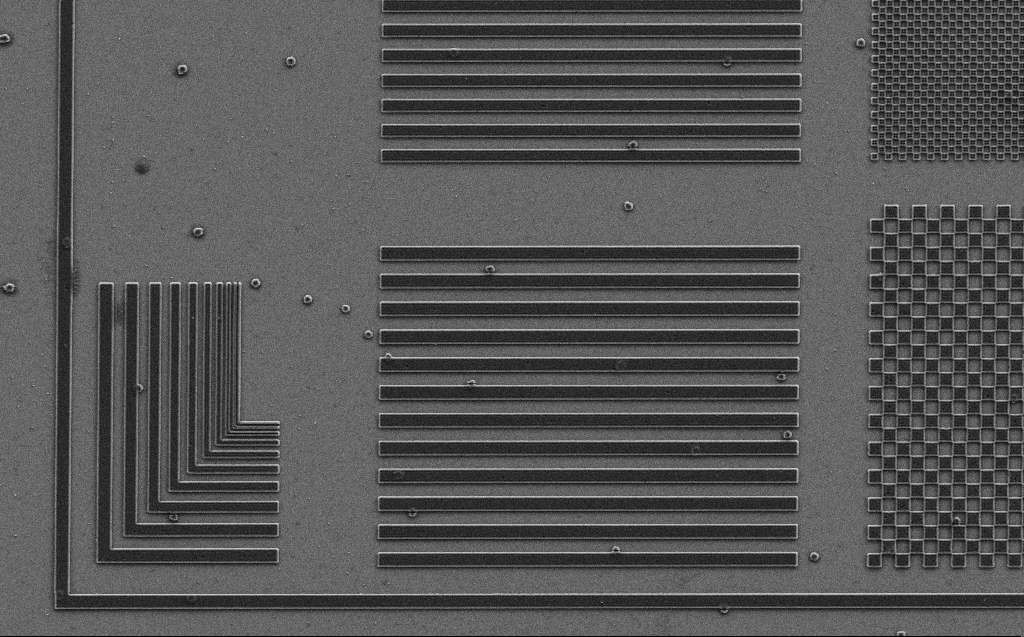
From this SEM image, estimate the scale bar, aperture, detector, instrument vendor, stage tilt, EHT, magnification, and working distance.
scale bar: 10000 nm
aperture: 30 µm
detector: SE2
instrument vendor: Zeiss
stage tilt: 0°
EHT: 3 kV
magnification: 5.19 K X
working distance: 6 mm